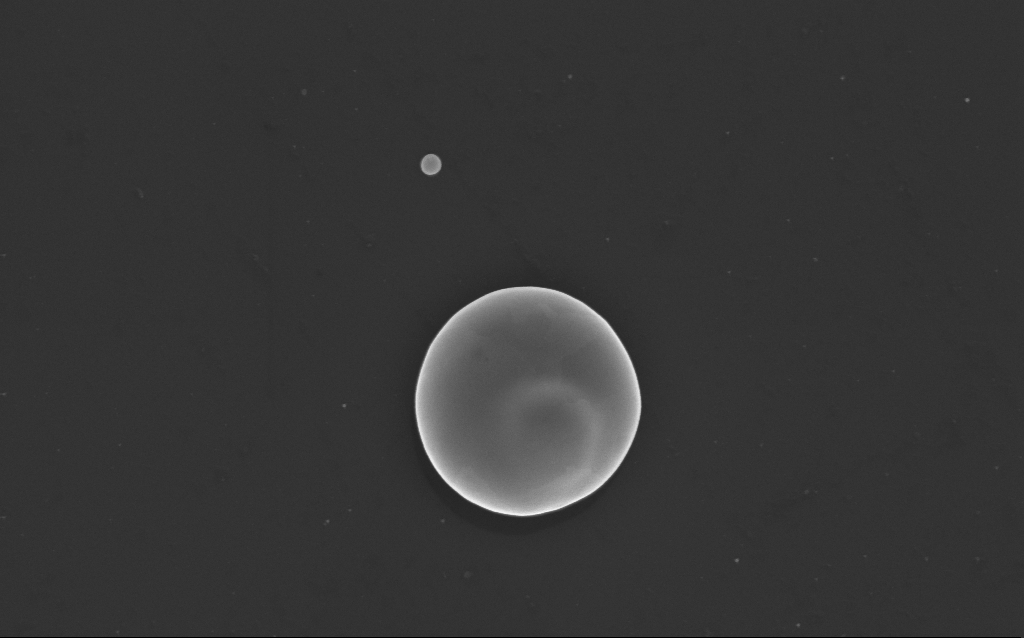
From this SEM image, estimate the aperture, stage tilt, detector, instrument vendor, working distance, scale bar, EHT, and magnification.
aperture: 30 µm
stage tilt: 0°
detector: InLens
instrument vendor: Zeiss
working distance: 3 mm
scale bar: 2000 nm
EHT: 10 kV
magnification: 35.25 K X